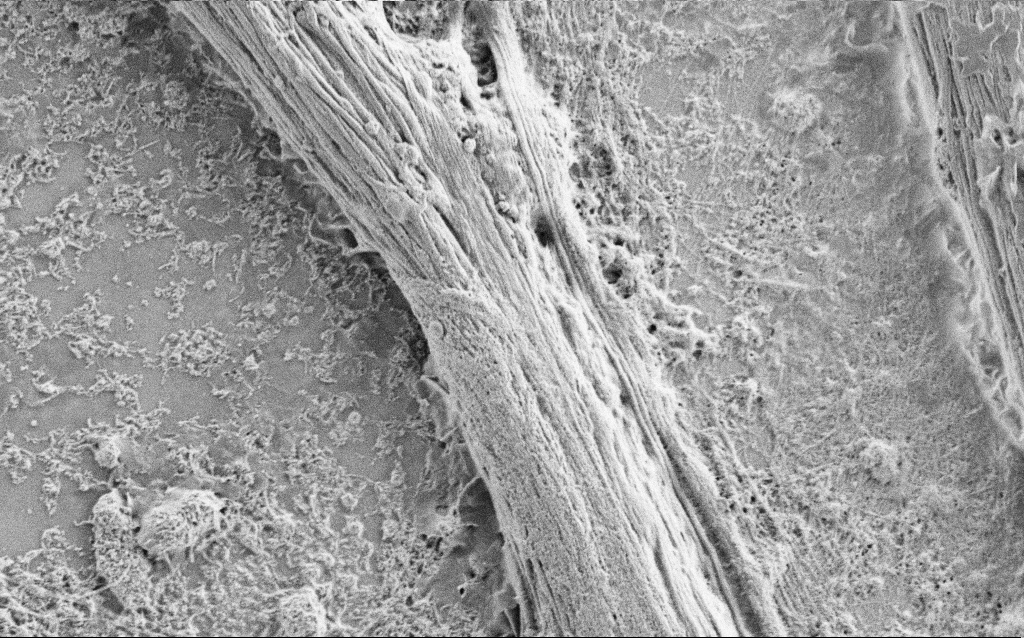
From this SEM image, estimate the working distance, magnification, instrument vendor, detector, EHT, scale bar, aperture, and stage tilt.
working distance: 4 mm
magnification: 10 K X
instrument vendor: Zeiss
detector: SE2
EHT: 0.9 kV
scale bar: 2000 nm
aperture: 30 µm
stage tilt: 0°